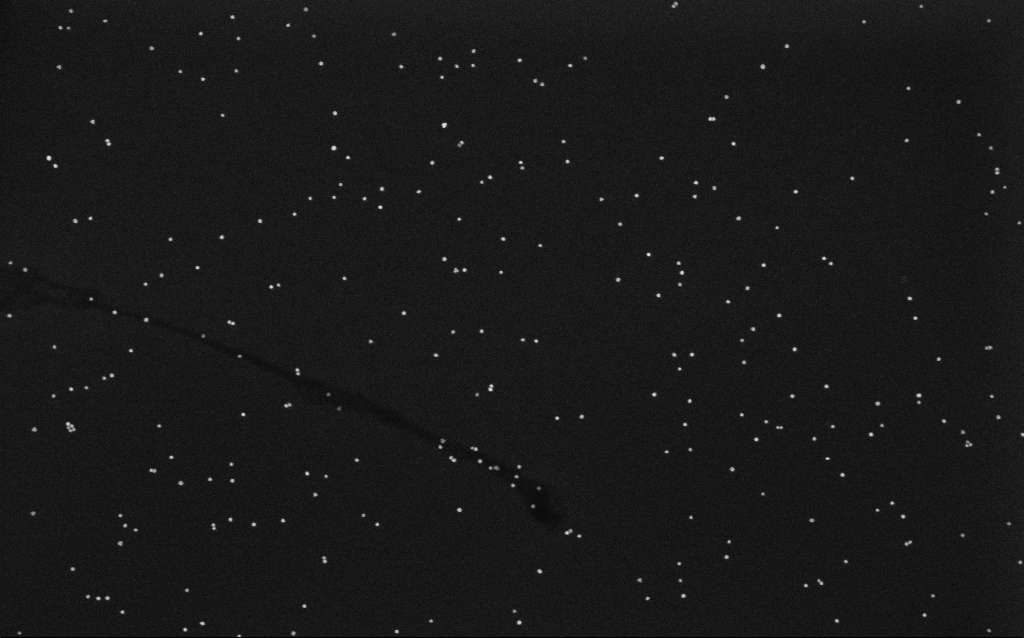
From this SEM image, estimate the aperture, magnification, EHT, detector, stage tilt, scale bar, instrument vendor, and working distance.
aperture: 30 µm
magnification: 100 K X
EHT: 10 kV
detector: InLens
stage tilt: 0°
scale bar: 200 nm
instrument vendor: Zeiss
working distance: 6.6 mm